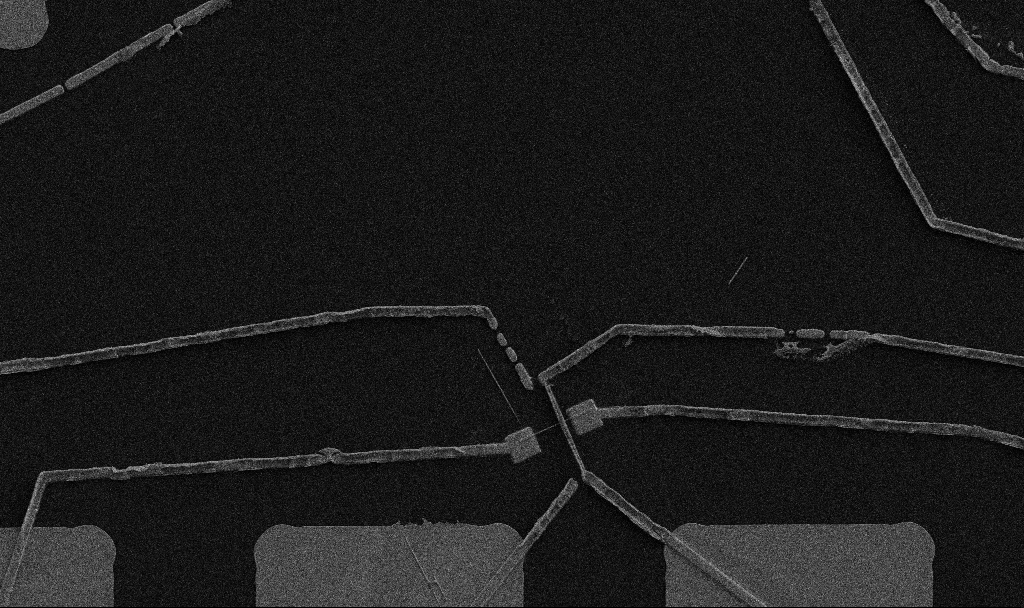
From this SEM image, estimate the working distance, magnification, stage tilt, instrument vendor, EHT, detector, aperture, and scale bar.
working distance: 10.7 mm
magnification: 5 K X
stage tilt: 0°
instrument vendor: Zeiss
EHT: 5 kV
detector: SE2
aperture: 30 µm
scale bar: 10000 nm